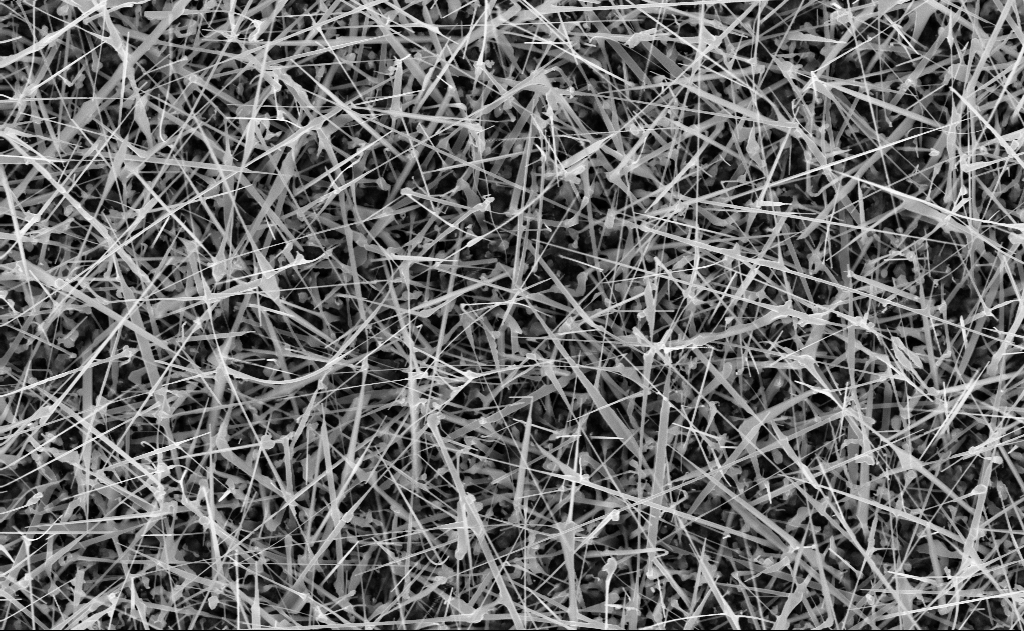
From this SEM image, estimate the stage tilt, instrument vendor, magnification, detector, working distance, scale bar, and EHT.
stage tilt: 0°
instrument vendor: Zeiss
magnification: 20 K X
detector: InLens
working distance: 10 mm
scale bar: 1000 nm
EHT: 10 kV